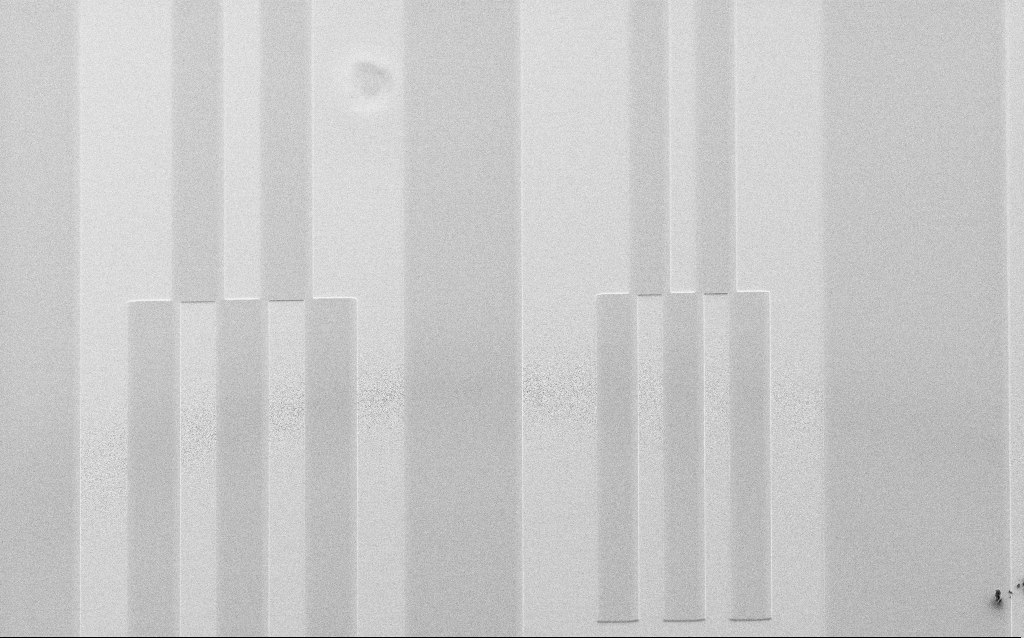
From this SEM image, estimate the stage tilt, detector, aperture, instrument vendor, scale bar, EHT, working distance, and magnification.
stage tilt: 45°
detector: SE2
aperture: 30 µm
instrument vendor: Zeiss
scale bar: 100000 nm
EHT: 3 kV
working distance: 8 mm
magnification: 0.407 K X